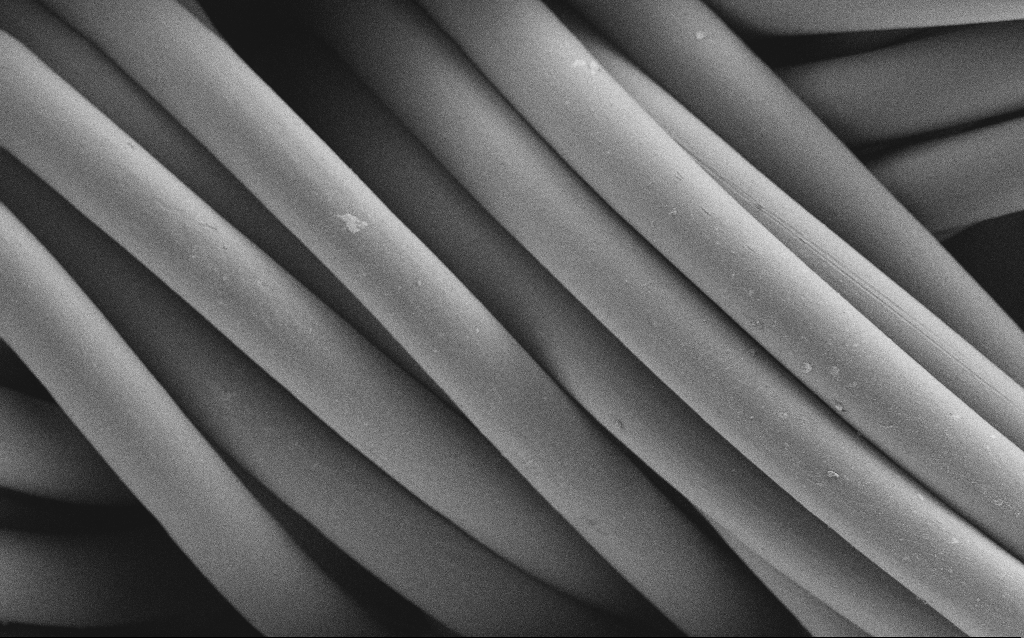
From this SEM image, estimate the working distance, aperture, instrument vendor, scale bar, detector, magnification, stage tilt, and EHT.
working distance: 4 mm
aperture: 30 µm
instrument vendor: Zeiss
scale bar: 20000 nm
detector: SE2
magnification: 1.43 K X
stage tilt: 0°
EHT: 1 kV